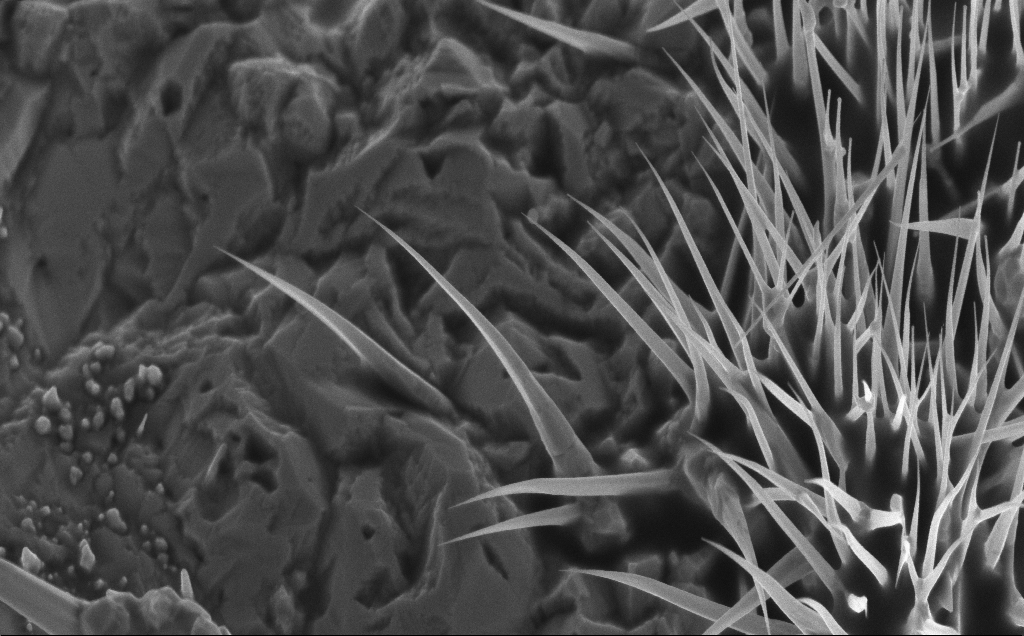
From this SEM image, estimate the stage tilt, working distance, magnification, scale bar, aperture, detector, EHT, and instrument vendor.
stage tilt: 30°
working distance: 8 mm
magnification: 25.43 K X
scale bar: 2000 nm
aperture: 30 µm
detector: InLens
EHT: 5 kV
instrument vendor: Zeiss